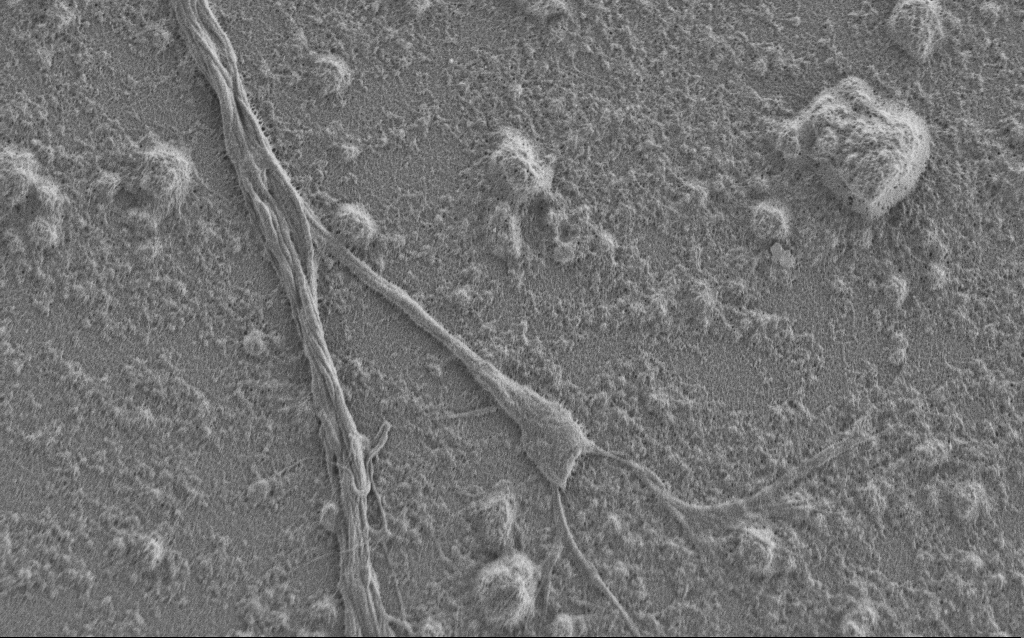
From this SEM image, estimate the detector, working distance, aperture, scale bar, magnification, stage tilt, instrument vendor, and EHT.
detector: SE2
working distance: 6 mm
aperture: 30 µm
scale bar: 2000 nm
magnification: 7.5 K X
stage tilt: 0°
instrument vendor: Zeiss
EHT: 1 kV